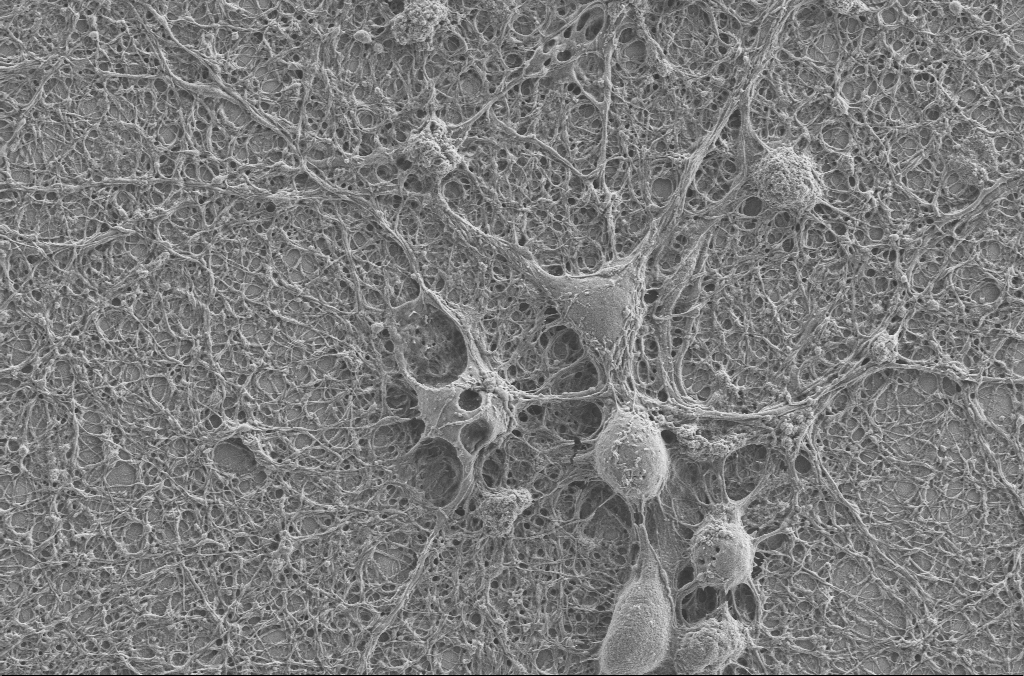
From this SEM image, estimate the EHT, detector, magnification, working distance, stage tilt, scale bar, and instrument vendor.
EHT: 2 kV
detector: SE2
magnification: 5 K X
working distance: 4 mm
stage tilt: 0°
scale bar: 10000 nm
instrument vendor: Zeiss